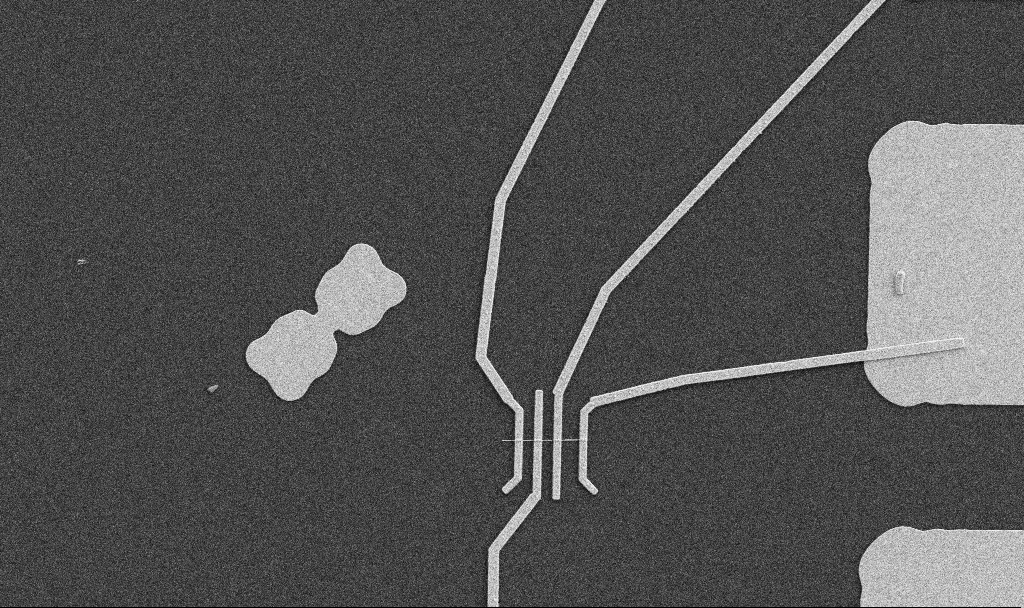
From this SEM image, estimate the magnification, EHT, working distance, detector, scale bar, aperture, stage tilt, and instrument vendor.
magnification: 5 K X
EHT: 5 kV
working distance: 10.7 mm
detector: SE2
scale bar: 10000 nm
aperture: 30 µm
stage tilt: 0°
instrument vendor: Zeiss